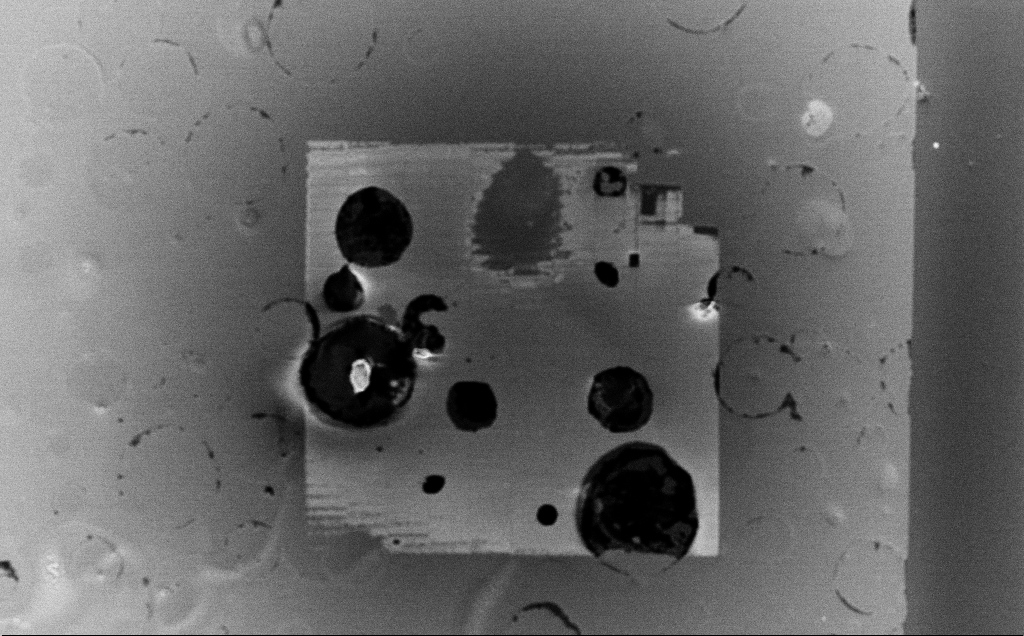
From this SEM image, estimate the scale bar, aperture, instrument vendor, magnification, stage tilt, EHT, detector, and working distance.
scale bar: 100000 nm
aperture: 30 µm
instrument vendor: Zeiss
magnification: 0.508 K X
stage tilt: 0°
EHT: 5 kV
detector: InLens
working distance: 2.6 mm